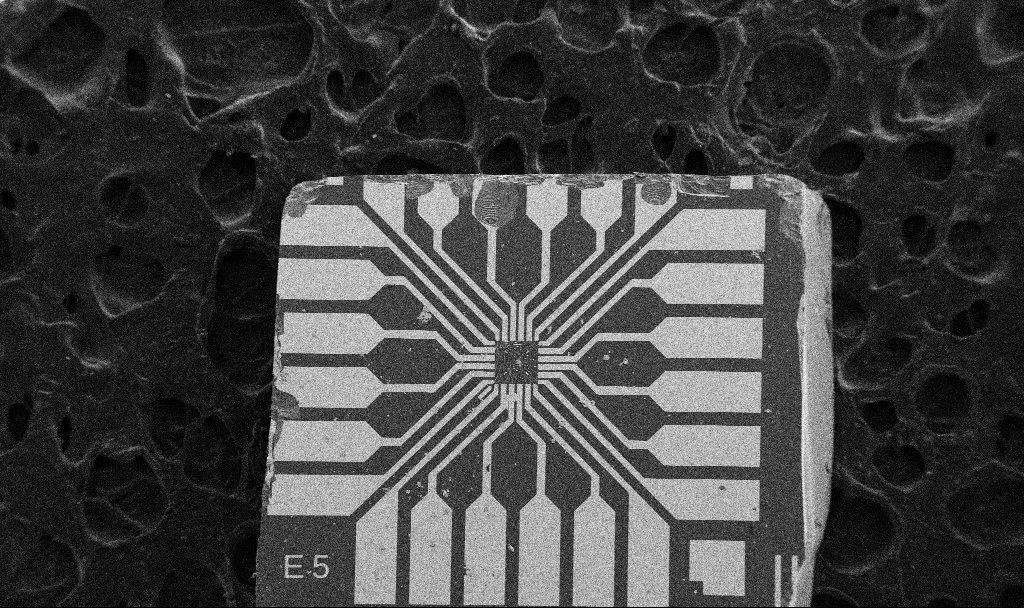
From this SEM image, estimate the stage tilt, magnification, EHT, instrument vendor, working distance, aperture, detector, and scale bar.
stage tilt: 0°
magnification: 0.1 K X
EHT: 5 kV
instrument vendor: Zeiss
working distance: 10.6 mm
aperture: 30 µm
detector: SE2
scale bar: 200000 nm